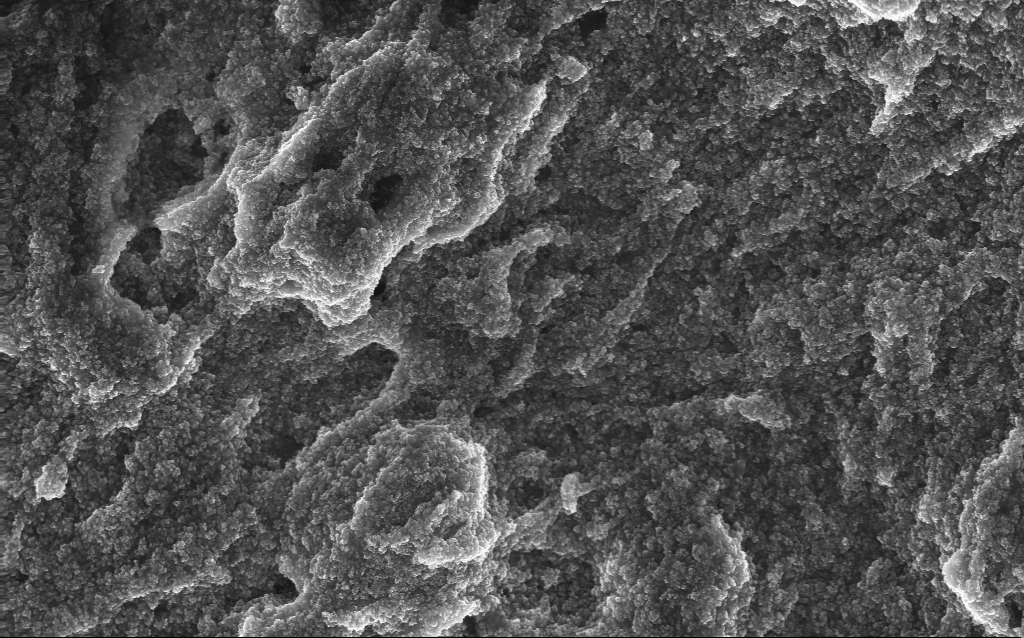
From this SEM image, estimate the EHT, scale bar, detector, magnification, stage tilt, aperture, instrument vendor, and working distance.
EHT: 10 kV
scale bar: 1000 nm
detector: InLens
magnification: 20.87 K X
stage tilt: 0°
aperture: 30 µm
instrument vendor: Zeiss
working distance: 2.6 mm